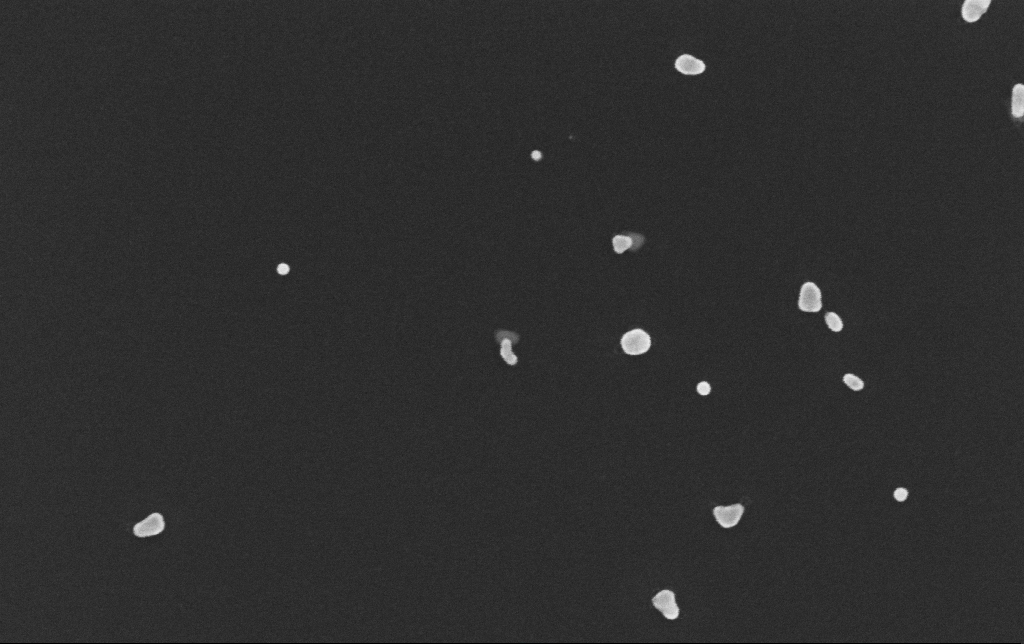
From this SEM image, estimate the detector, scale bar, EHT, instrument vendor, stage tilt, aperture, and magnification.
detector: InLens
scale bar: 200 nm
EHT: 10 kV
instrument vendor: Zeiss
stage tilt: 0°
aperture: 30 µm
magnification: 200 K X